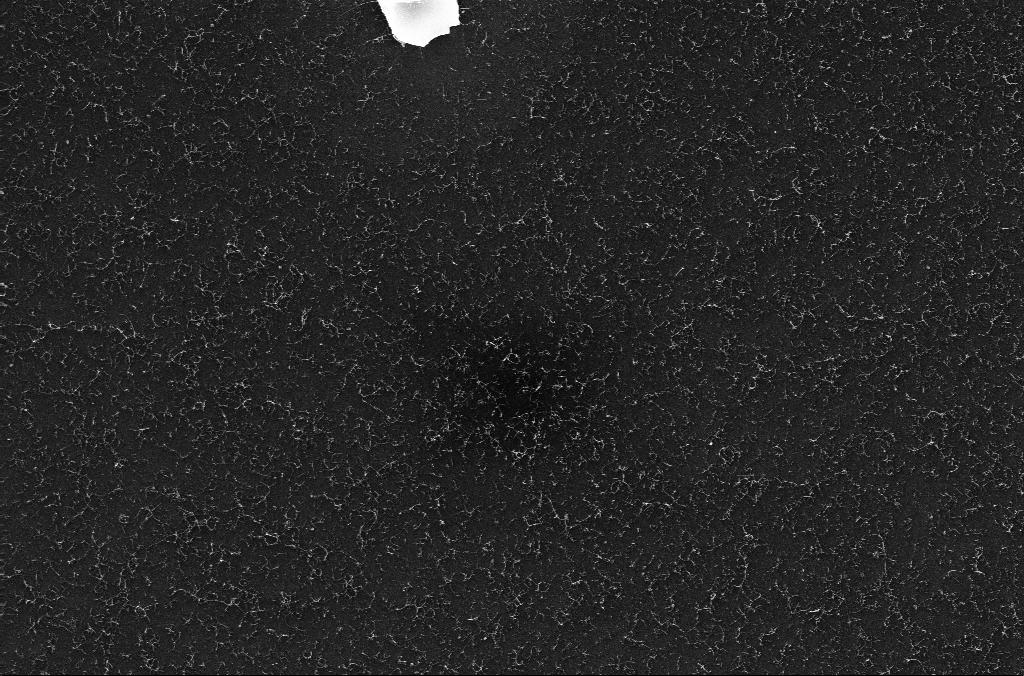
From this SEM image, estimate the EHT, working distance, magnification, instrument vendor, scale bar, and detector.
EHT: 10 kV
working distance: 3.2 mm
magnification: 17.02 K X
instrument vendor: Zeiss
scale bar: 1000 nm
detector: InLens